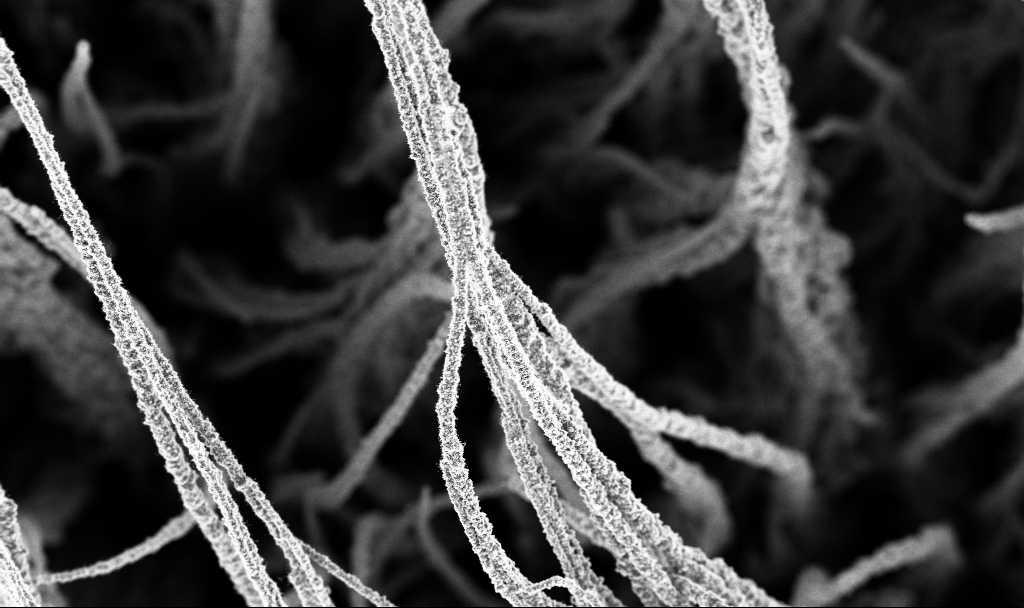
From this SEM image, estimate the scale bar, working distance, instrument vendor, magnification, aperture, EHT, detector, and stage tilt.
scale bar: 100000 nm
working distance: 2.7 mm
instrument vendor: Zeiss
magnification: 0.5 K X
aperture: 30 µm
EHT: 3 kV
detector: InLens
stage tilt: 0°